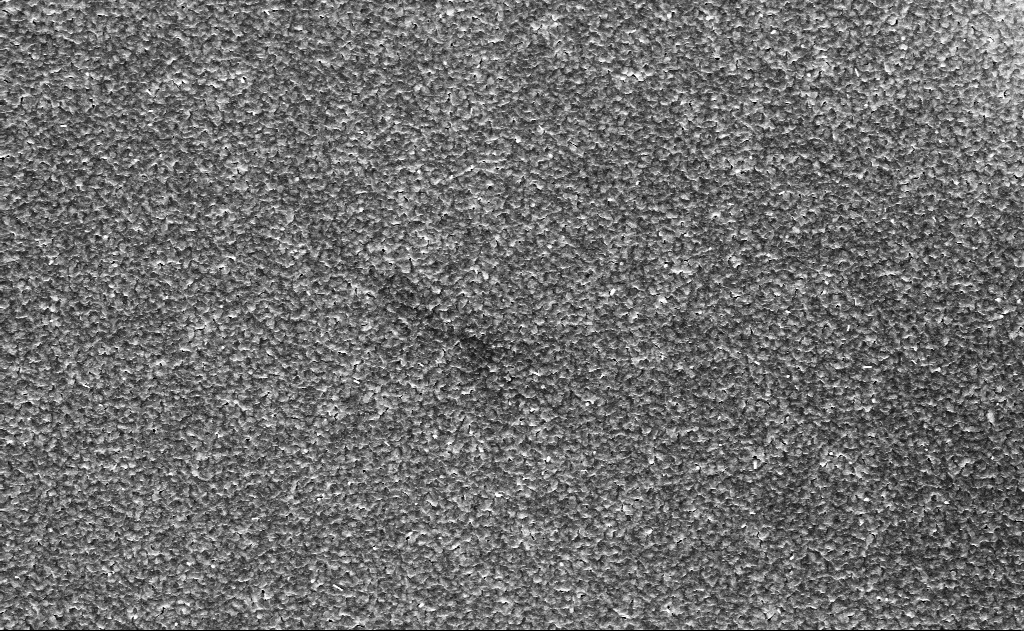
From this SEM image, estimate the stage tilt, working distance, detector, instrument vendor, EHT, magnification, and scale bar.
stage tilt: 0°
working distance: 11 mm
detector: InLens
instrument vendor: Zeiss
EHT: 10 kV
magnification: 10 K X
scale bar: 2000 nm